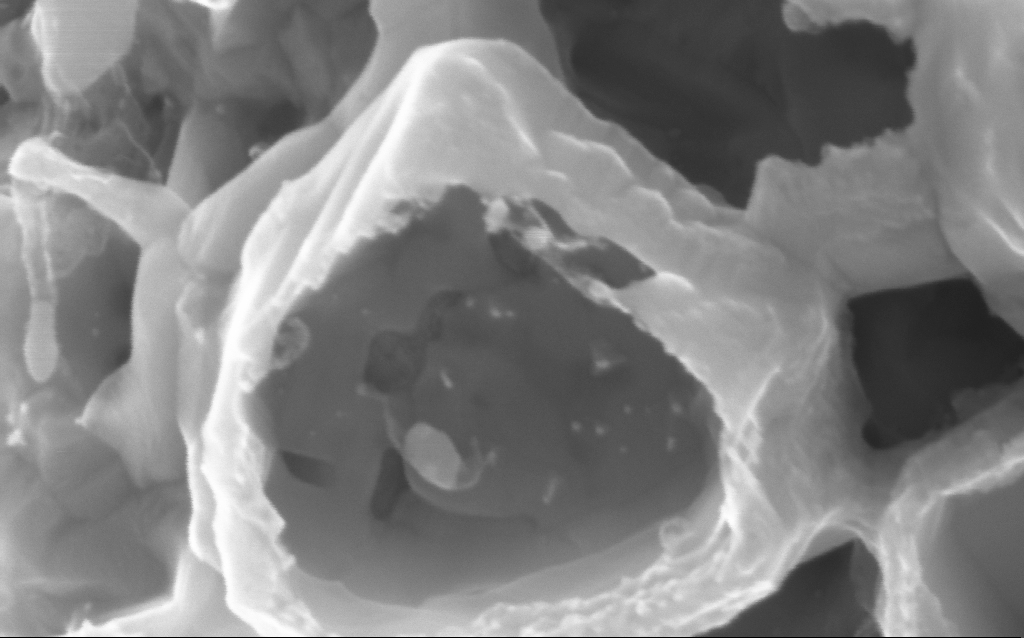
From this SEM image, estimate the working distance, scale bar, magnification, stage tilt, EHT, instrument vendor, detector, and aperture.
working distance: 2 mm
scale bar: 100 nm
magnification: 452.21 K X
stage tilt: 0°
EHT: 10 kV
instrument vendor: Zeiss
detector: InLens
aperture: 30 µm